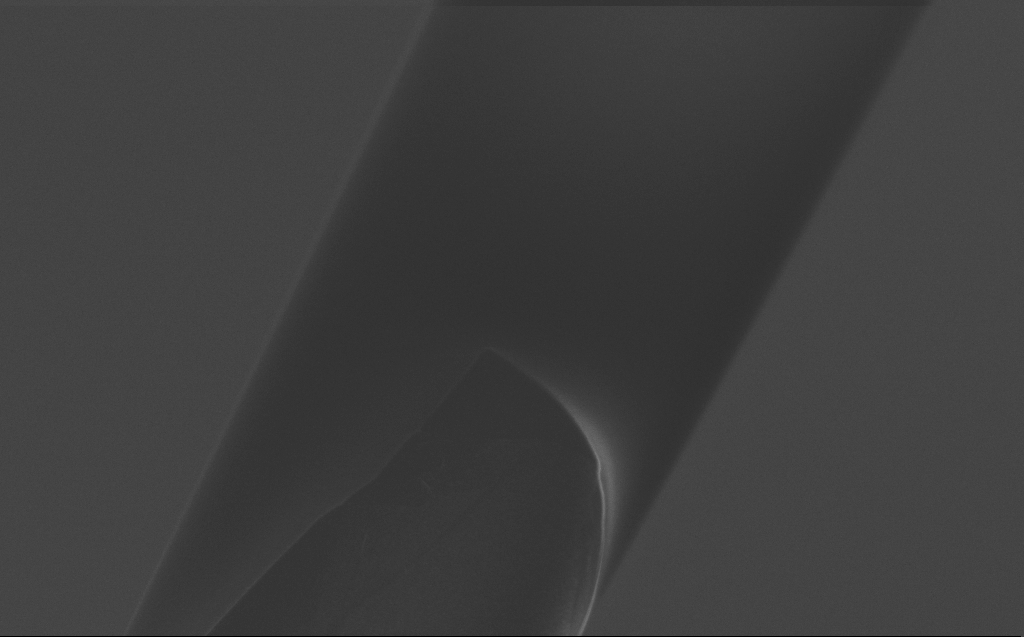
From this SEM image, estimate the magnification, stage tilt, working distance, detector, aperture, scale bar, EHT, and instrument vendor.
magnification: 6.21 K X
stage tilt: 45°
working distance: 6 mm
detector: InLens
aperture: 30 µm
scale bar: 10000 nm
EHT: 5 kV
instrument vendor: Zeiss